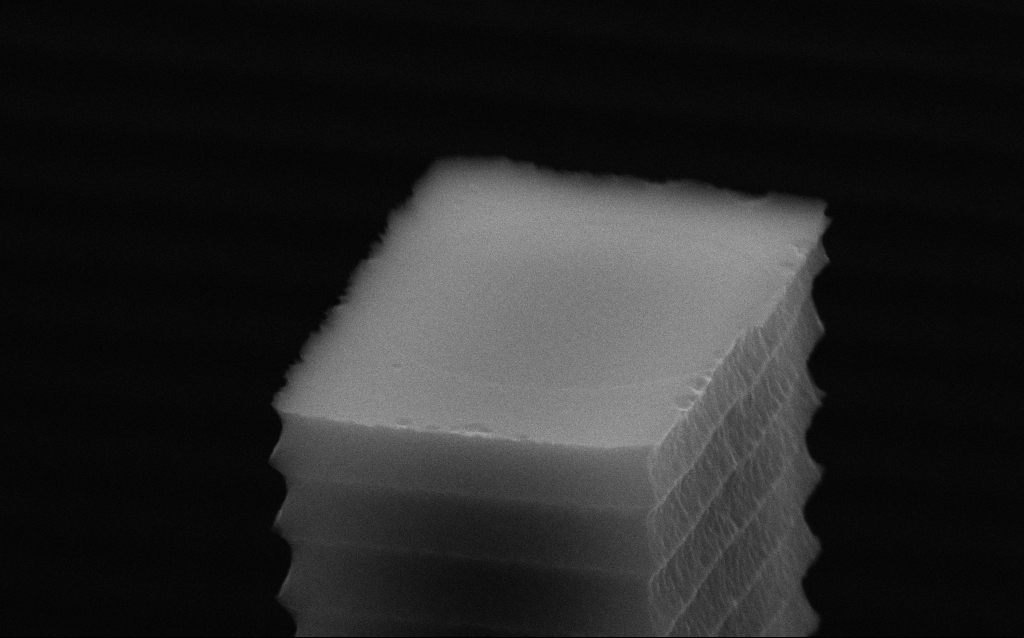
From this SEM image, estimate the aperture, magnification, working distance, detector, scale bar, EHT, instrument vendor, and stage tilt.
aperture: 30 µm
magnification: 83.12 K X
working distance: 7.2 mm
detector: SE2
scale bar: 200 nm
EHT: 10 kV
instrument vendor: Zeiss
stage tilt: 70°